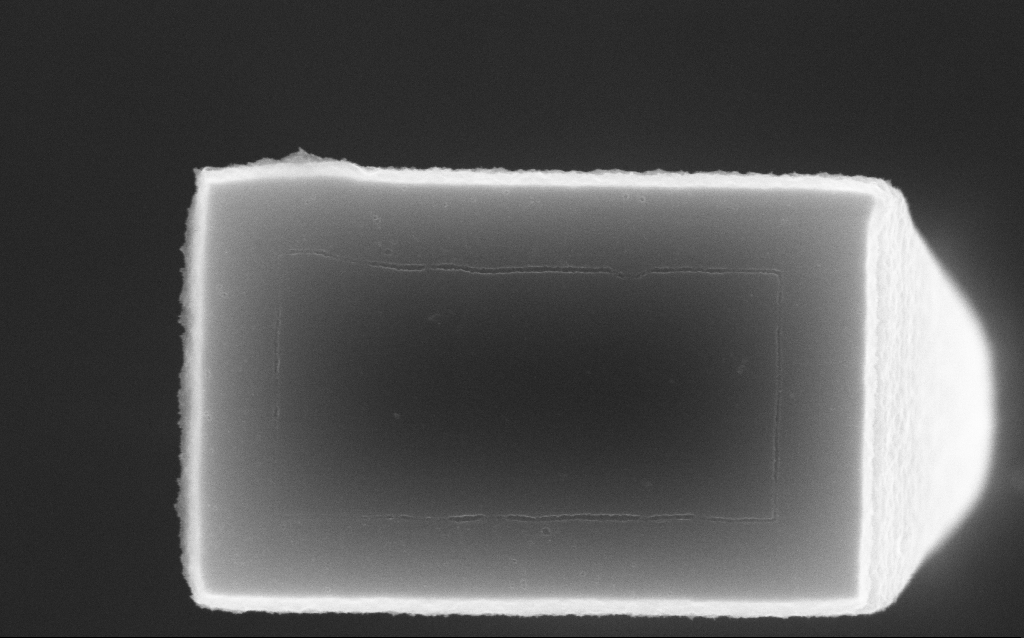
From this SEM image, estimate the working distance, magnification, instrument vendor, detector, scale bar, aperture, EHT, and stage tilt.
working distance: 8.3 mm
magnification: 89.21 K X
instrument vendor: Zeiss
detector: InLens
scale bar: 200 nm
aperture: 30 µm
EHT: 10 kV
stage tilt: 0°